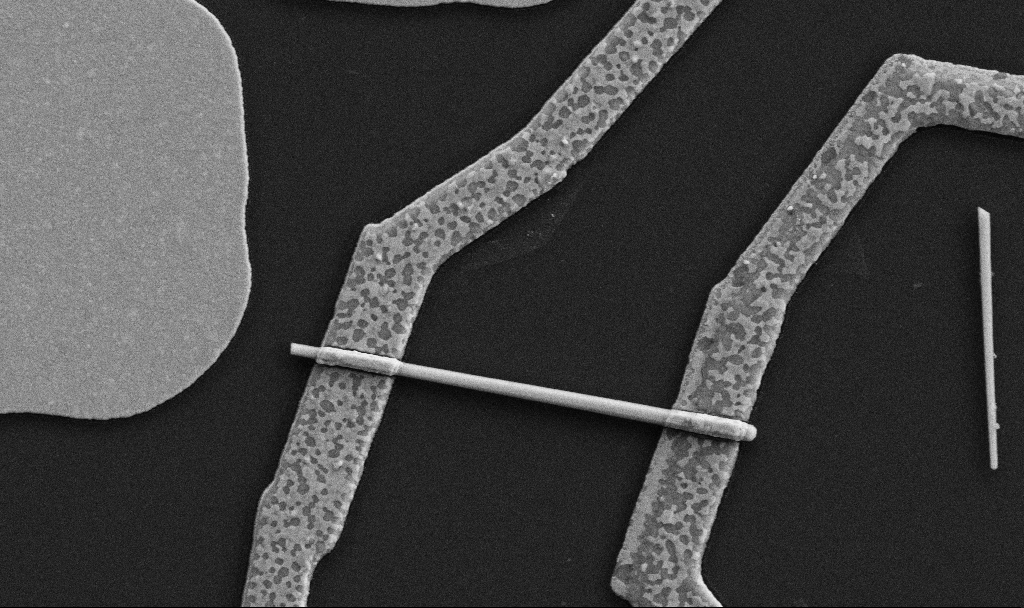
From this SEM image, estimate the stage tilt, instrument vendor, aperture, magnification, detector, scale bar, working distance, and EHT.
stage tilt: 0°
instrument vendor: Zeiss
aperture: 30 µm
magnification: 30 K X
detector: SE2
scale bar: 1000 nm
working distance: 8.7 mm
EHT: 5 kV